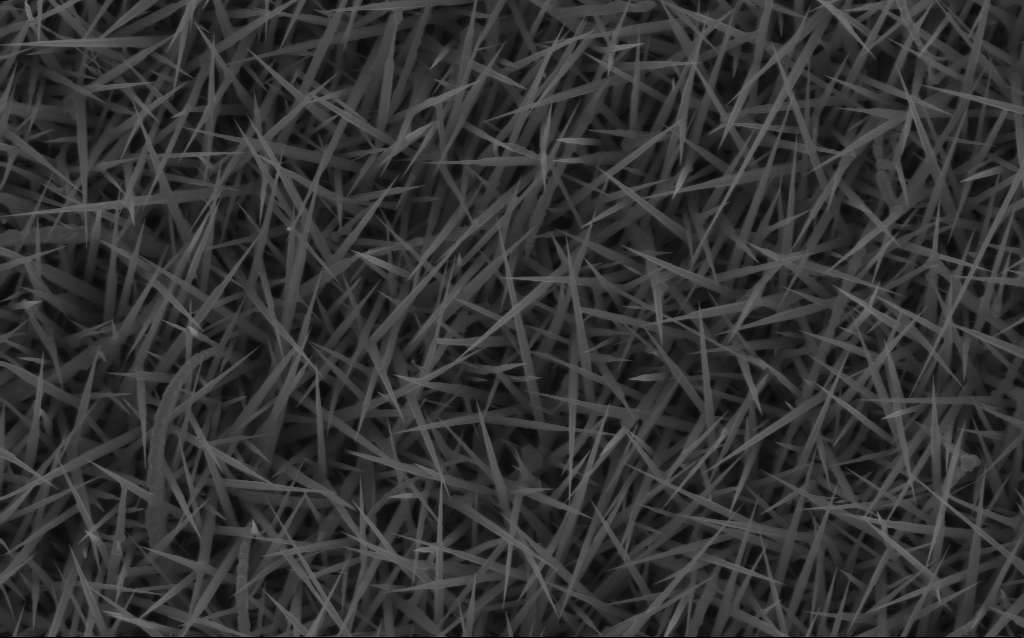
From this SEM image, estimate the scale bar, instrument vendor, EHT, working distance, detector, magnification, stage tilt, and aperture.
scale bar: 2000 nm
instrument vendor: Zeiss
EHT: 10 kV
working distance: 6 mm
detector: InLens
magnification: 20 K X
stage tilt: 0°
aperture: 30 µm